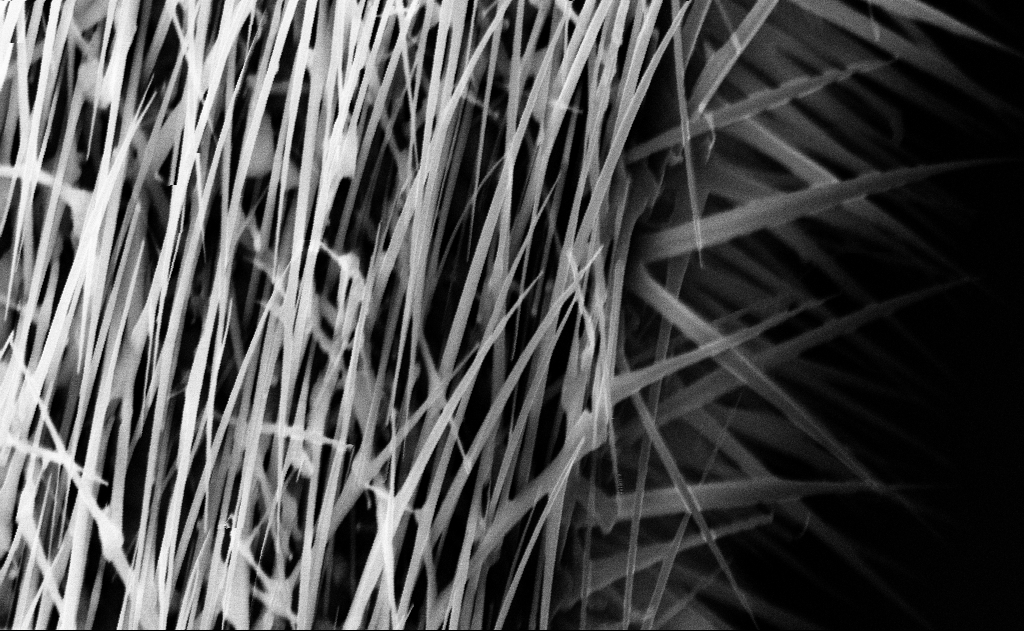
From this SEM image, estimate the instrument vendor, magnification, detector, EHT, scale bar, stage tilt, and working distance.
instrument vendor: Zeiss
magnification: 40 K X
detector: InLens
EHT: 10 kV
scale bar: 1000 nm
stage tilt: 0°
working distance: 16 mm